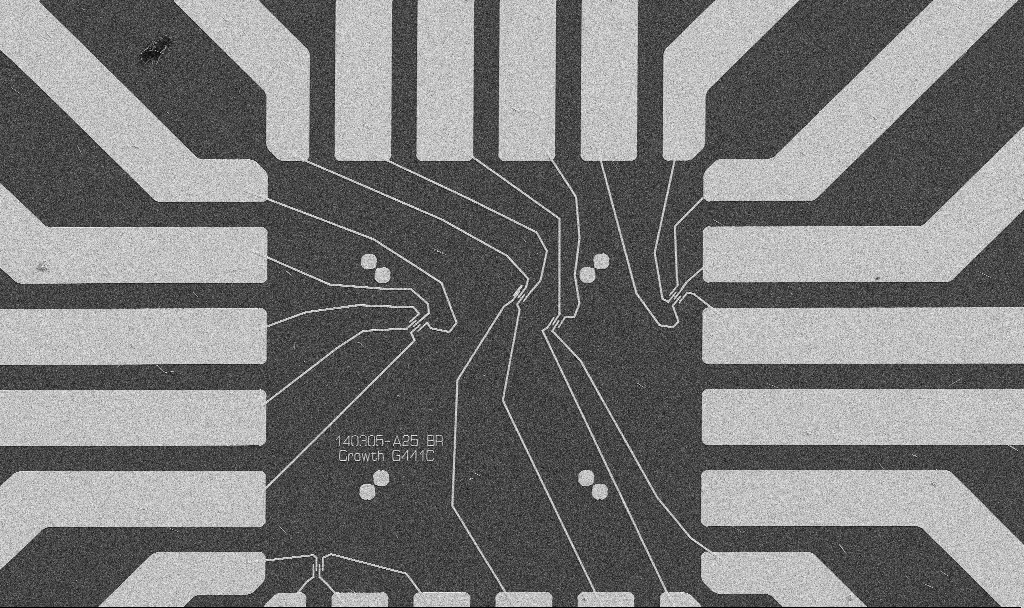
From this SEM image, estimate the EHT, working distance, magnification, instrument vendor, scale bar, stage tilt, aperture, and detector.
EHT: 5 kV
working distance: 10.7 mm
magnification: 1 K X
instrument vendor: Zeiss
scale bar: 20000 nm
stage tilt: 0°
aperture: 30 µm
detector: SE2